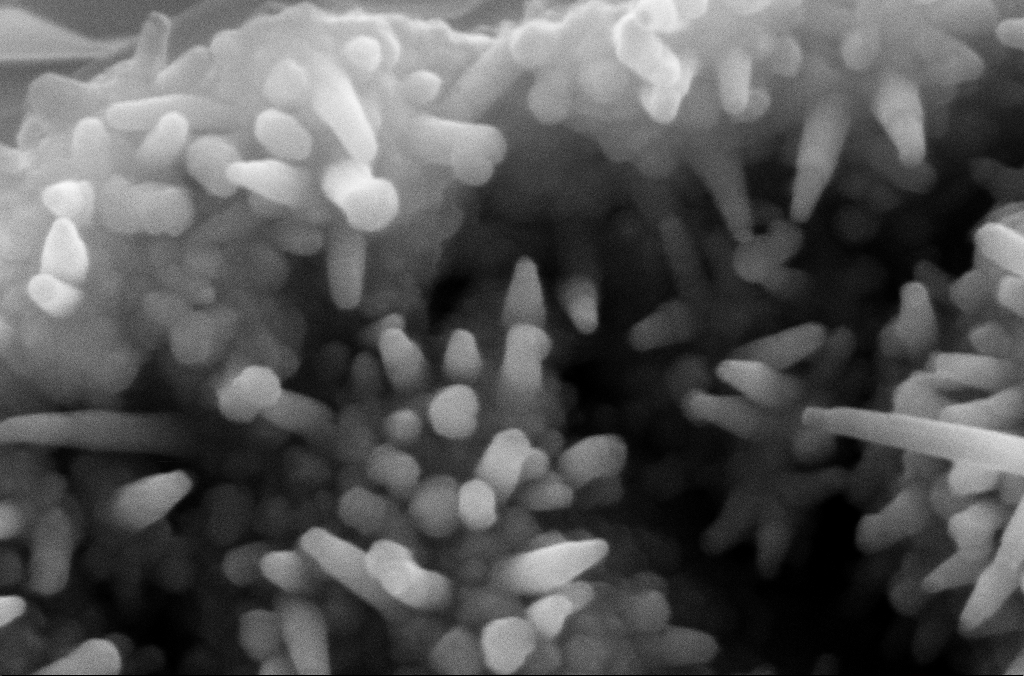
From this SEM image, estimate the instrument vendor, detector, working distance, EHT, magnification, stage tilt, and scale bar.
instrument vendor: Zeiss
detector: SE2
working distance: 5.2 mm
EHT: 10 kV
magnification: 244.06 K X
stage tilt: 0.1°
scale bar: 200 nm